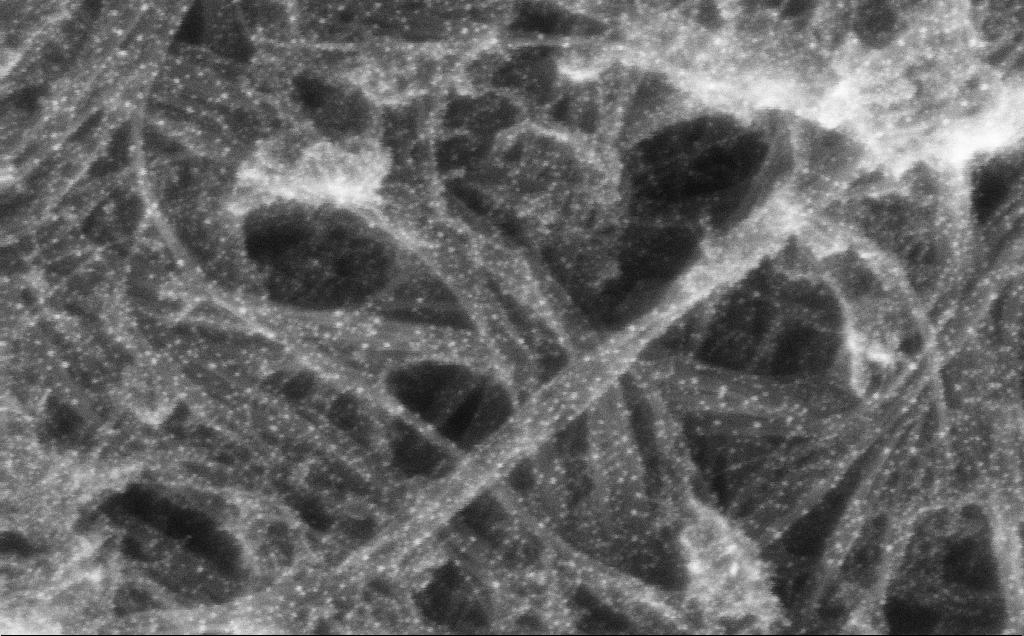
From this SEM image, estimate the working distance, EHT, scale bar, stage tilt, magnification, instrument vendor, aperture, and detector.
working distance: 3 mm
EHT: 10 kV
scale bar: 100 nm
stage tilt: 0°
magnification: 344.88 K X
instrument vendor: Zeiss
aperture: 30 µm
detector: InLens